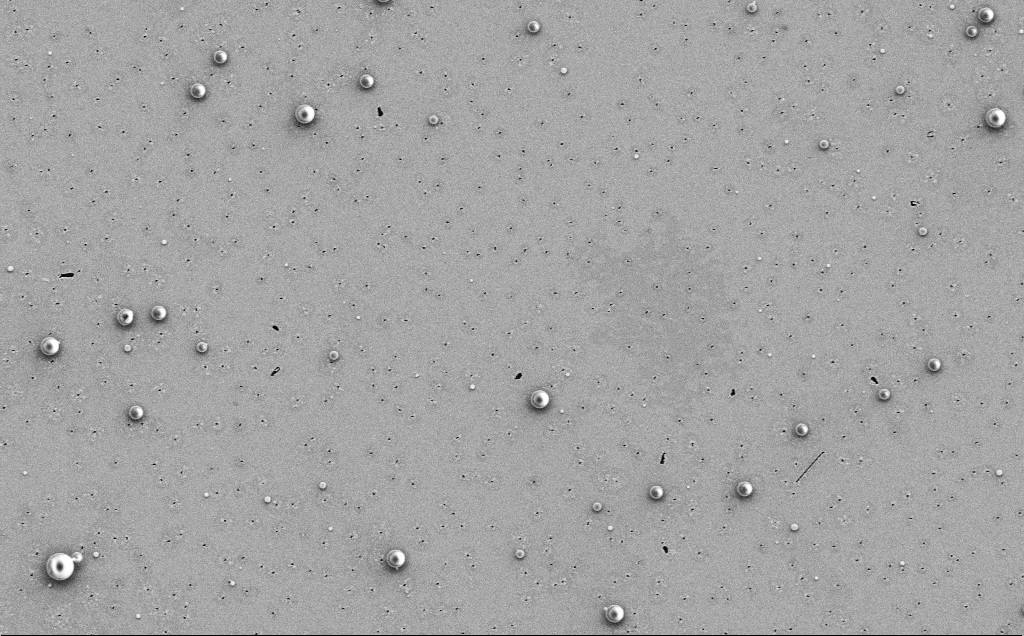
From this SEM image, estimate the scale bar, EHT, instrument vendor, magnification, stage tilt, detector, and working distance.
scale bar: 10000 nm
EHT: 5 kV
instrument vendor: Zeiss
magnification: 4.2 K X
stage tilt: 0°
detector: SE2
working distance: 12 mm